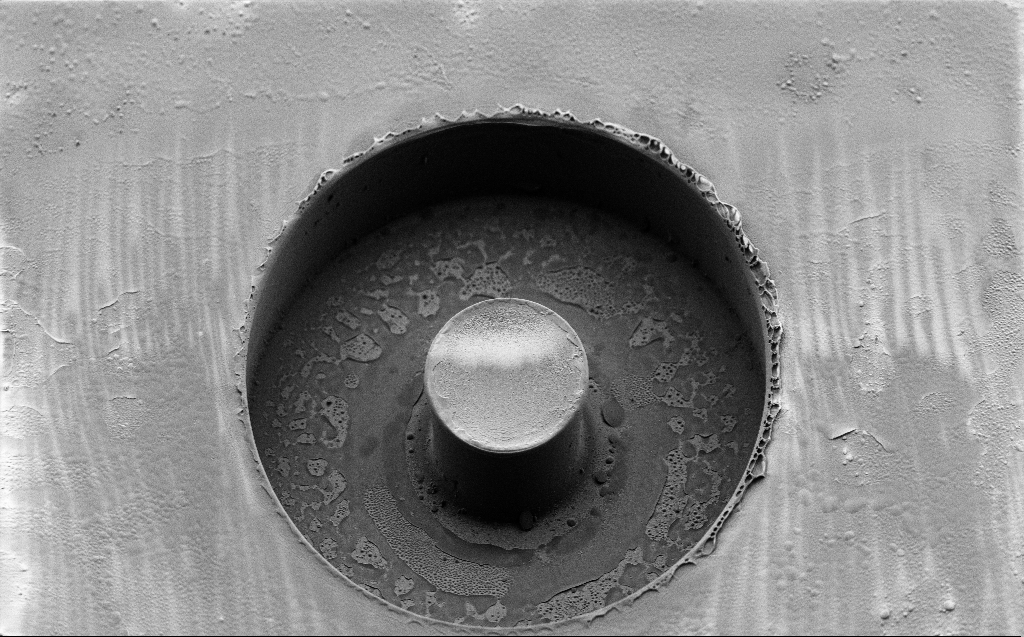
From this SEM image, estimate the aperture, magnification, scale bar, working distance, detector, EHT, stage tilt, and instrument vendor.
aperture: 30 µm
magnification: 1.48 K X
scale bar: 20000 nm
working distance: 7 mm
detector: SE2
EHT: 2 kV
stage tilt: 45°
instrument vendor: Zeiss